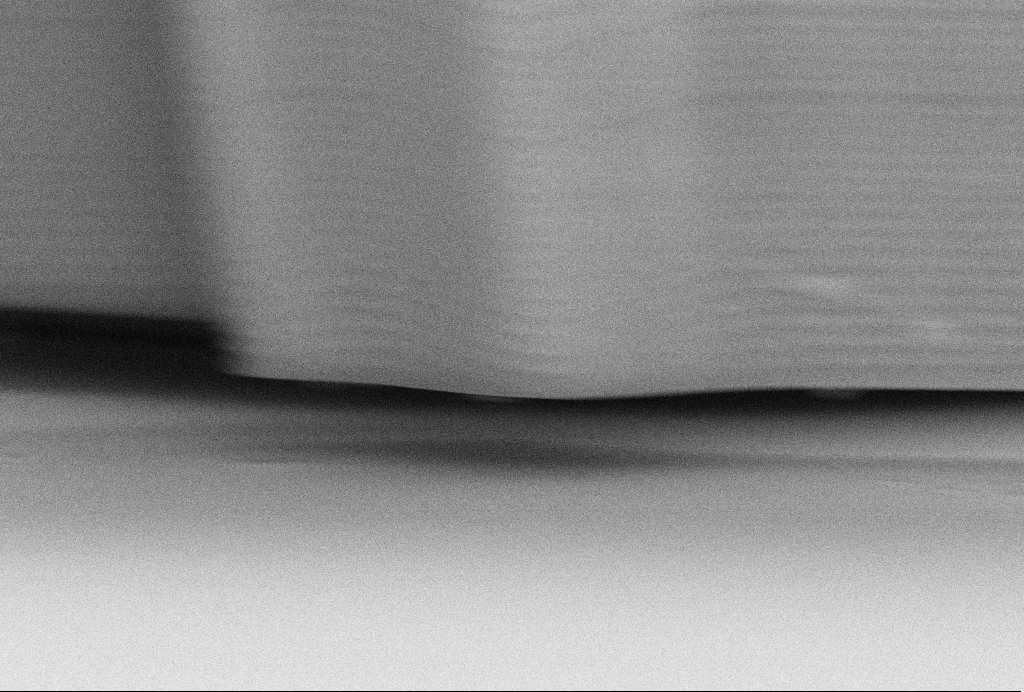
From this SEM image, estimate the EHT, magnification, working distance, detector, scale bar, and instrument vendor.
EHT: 10 kV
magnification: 26.08 K X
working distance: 8 mm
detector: SE2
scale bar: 2000 nm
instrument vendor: Zeiss